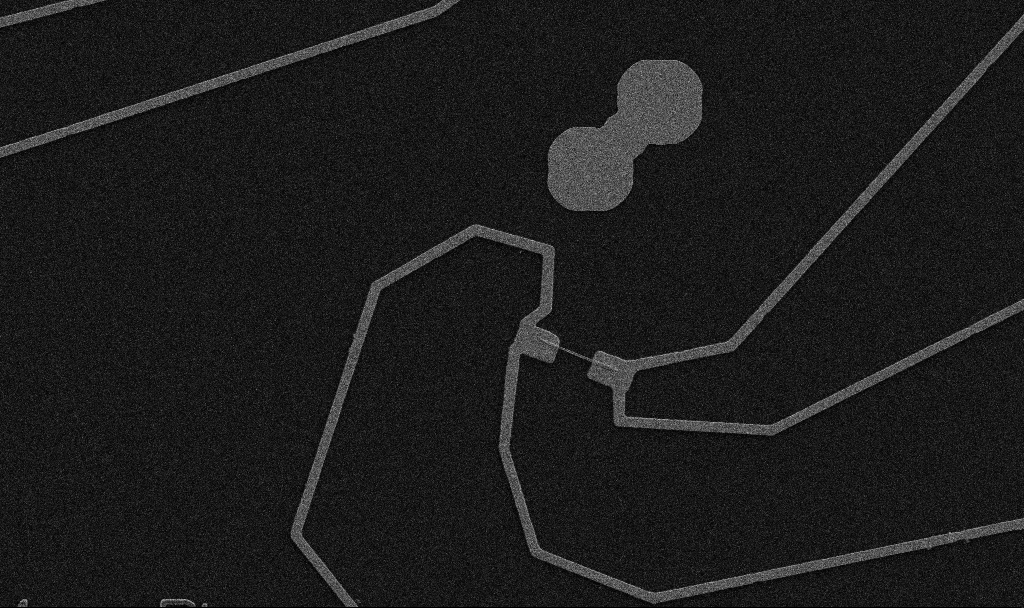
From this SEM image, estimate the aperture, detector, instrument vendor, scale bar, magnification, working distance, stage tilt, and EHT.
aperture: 30 µm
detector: SE2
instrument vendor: Zeiss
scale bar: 10000 nm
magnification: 5 K X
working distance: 10.7 mm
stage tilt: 0°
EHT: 5 kV